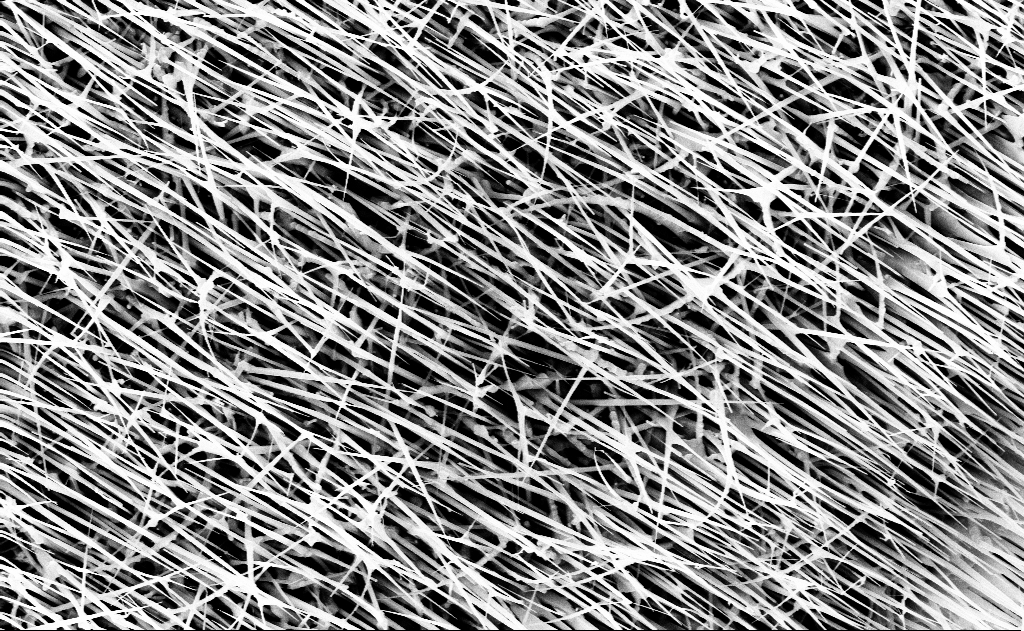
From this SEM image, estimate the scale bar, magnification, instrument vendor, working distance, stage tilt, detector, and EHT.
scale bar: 2000 nm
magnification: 20 K X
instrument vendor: Zeiss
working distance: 13 mm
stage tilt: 0°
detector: InLens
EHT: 10 kV